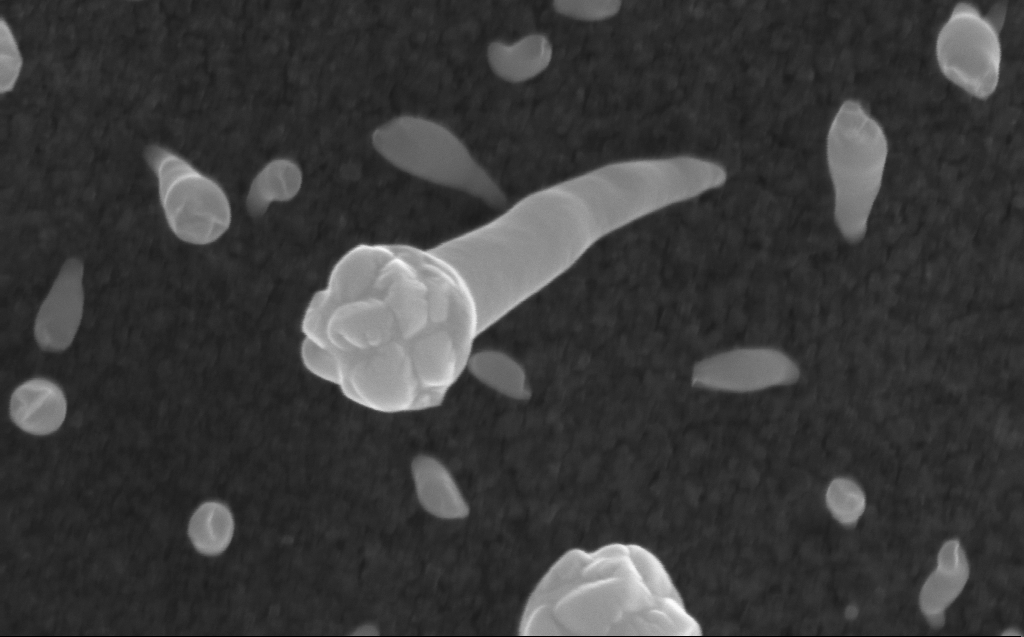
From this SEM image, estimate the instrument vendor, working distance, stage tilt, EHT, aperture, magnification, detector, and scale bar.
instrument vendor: Zeiss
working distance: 3 mm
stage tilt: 0°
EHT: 10 kV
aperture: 30 µm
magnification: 200 K X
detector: InLens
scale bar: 100 nm